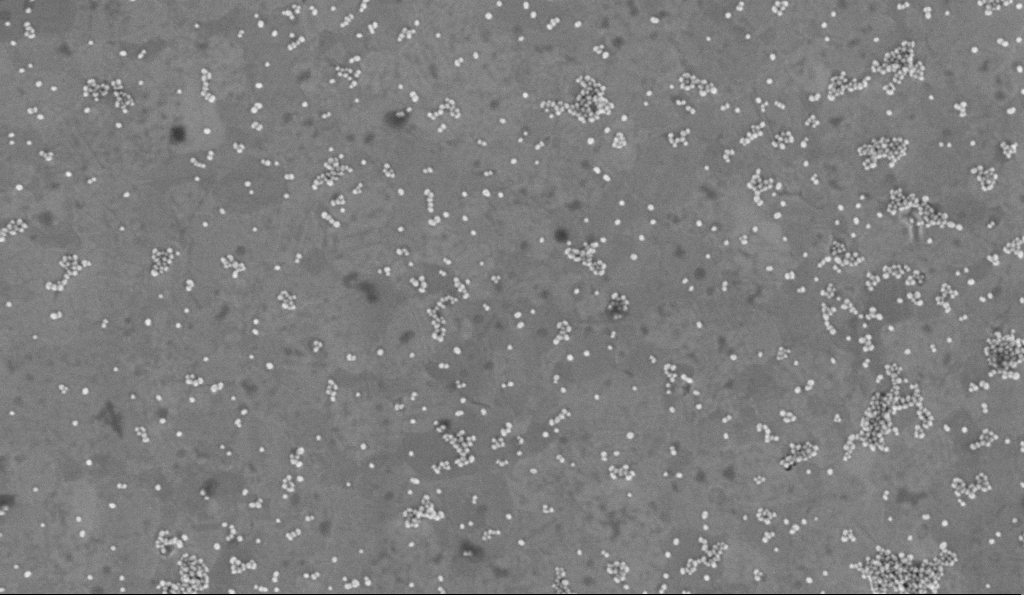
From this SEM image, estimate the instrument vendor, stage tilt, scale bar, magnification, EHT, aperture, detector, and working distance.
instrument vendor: Zeiss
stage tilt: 0°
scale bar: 200 nm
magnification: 75 K X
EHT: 2 kV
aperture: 30 µm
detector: InLens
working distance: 3.2 mm